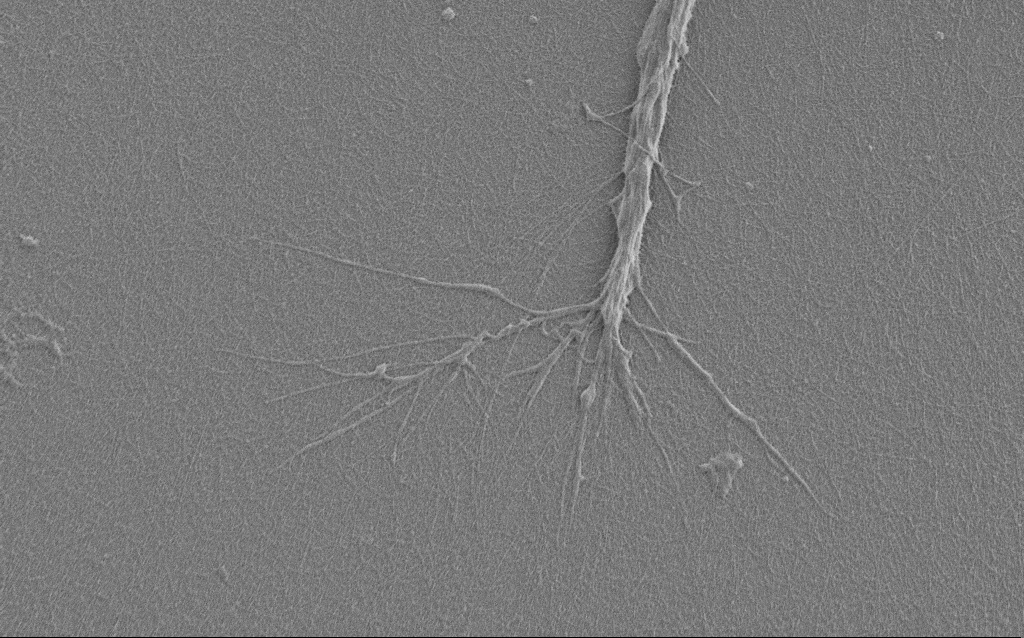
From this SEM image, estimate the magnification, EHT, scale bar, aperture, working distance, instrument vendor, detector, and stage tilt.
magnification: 7.5 K X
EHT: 1 kV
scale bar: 2000 nm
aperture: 30 µm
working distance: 6 mm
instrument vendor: Zeiss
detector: SE2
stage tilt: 0°